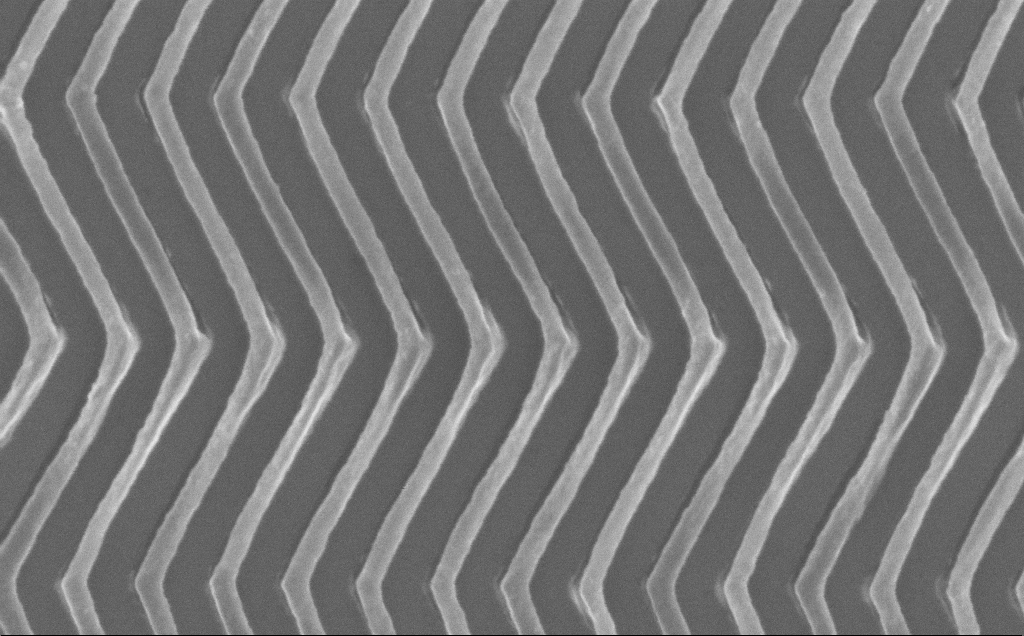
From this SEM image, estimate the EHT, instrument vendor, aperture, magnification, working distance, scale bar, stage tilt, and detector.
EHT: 10 kV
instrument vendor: Zeiss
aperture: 30 µm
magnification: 89.95 K X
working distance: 7 mm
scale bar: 200 nm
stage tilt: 0°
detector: InLens